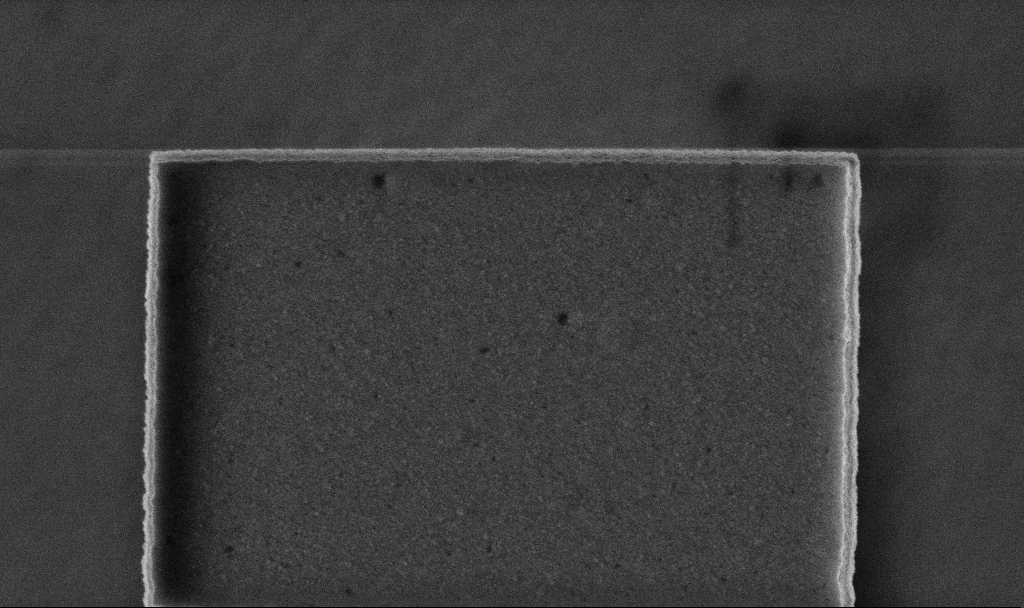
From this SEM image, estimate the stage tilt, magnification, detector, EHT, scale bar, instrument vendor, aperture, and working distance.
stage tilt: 0°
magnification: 57.48 K X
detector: InLens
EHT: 5 kV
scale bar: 1000 nm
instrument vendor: Zeiss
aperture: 30 µm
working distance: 3.2 mm